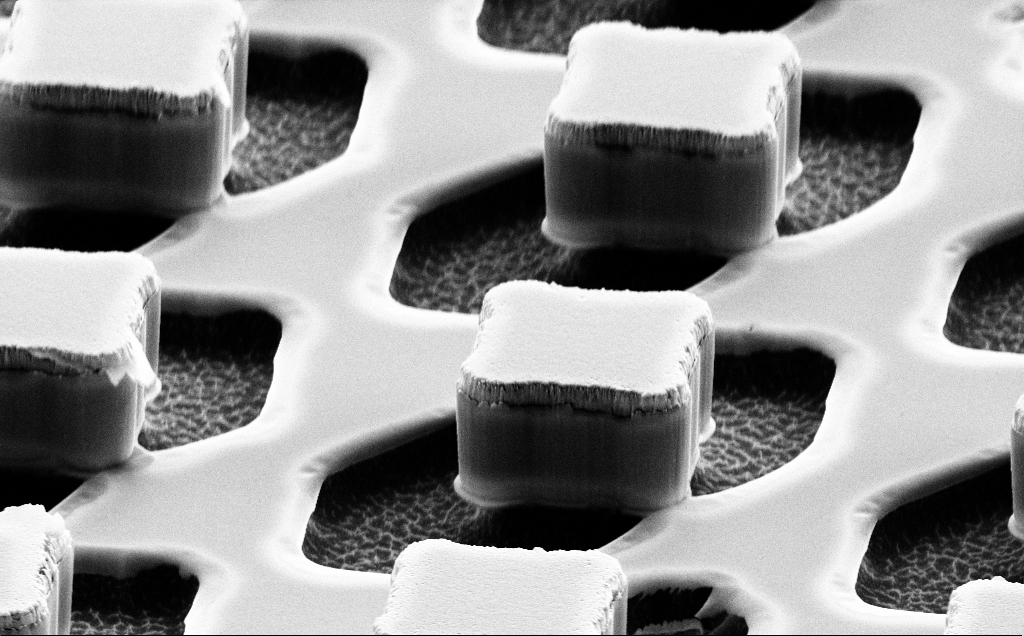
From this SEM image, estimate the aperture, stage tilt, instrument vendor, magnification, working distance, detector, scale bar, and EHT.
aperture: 30 µm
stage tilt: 61.7°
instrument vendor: Zeiss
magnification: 10.27 K X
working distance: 12 mm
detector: SE2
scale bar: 2000 nm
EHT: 10 kV